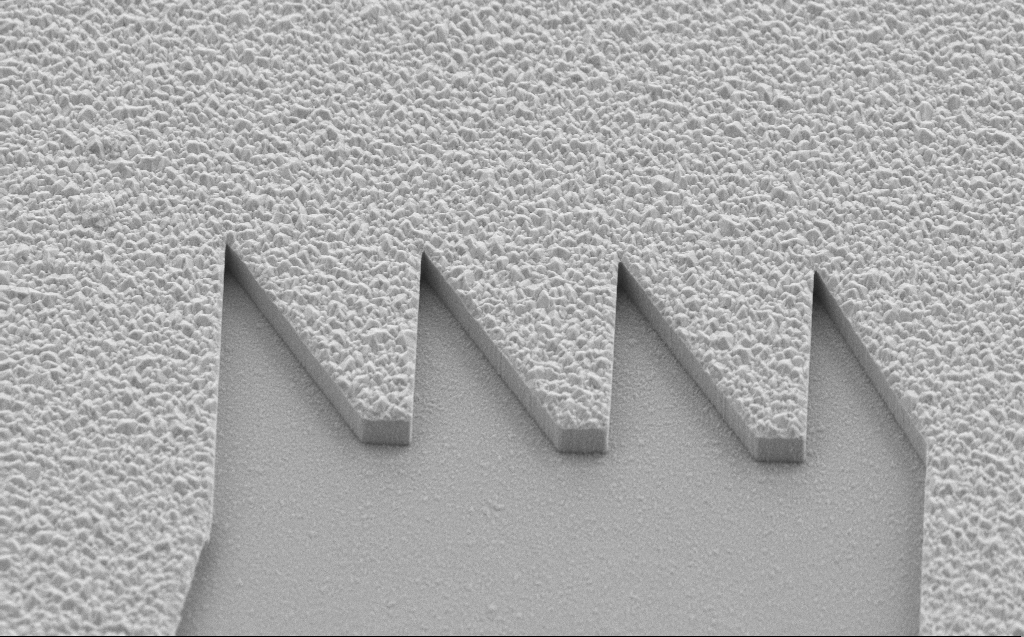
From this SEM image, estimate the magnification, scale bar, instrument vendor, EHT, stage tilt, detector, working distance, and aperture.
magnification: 6.3 K X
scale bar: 10000 nm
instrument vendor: Zeiss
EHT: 10 kV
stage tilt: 45°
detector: SE2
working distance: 5 mm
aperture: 30 µm